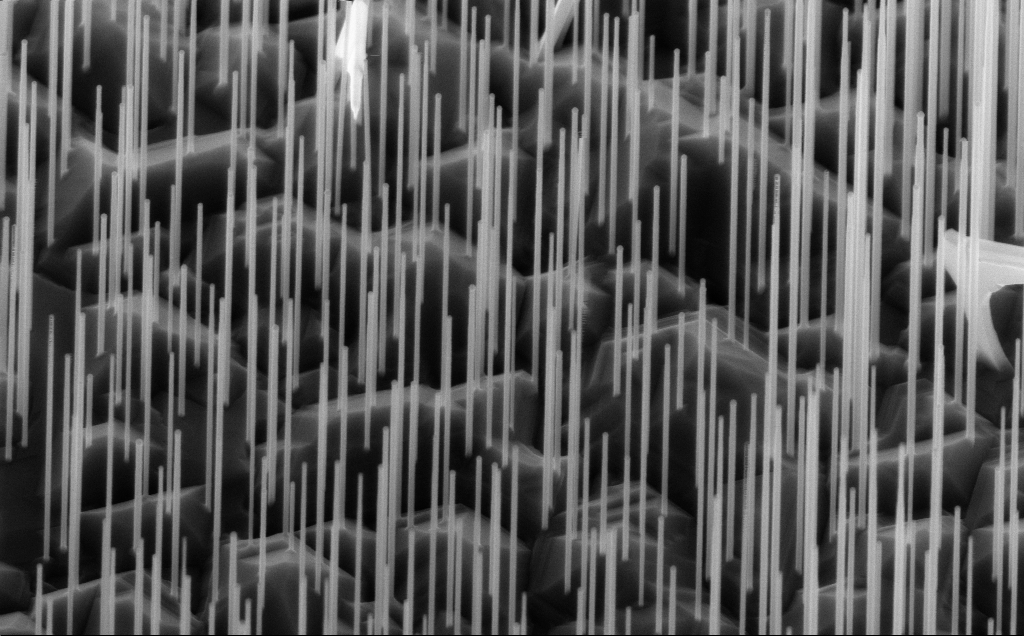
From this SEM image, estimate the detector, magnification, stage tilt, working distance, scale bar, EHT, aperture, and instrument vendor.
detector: InLens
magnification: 40 K X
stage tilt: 45°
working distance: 6 mm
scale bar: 1000 nm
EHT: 10 kV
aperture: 30 µm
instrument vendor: Zeiss